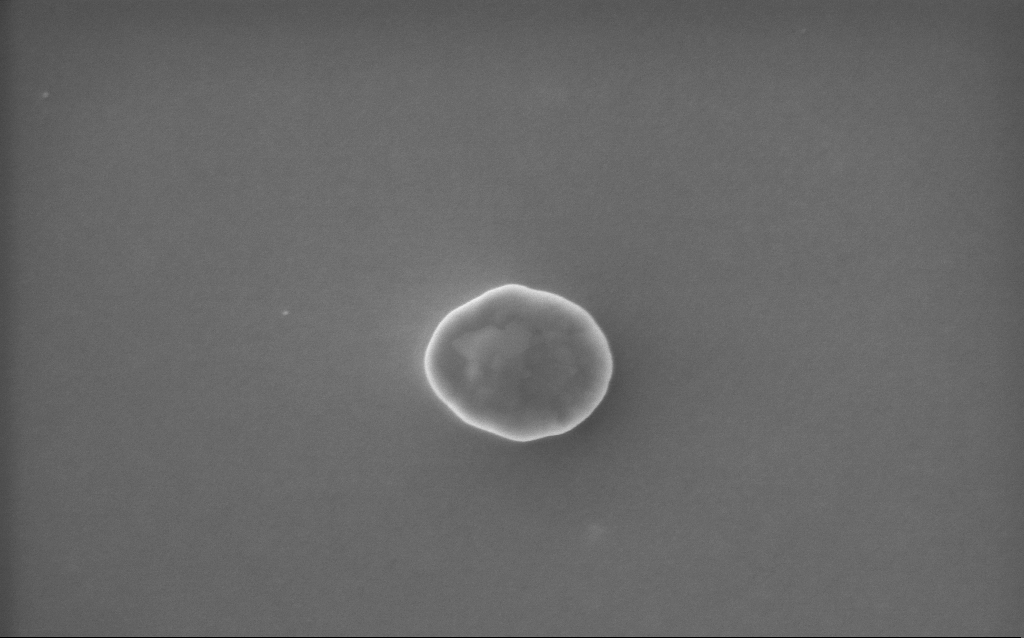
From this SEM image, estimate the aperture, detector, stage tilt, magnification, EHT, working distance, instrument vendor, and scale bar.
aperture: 30 µm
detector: InLens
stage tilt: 0°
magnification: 75.1 K X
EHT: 5 kV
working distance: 2 mm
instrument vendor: Zeiss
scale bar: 200 nm